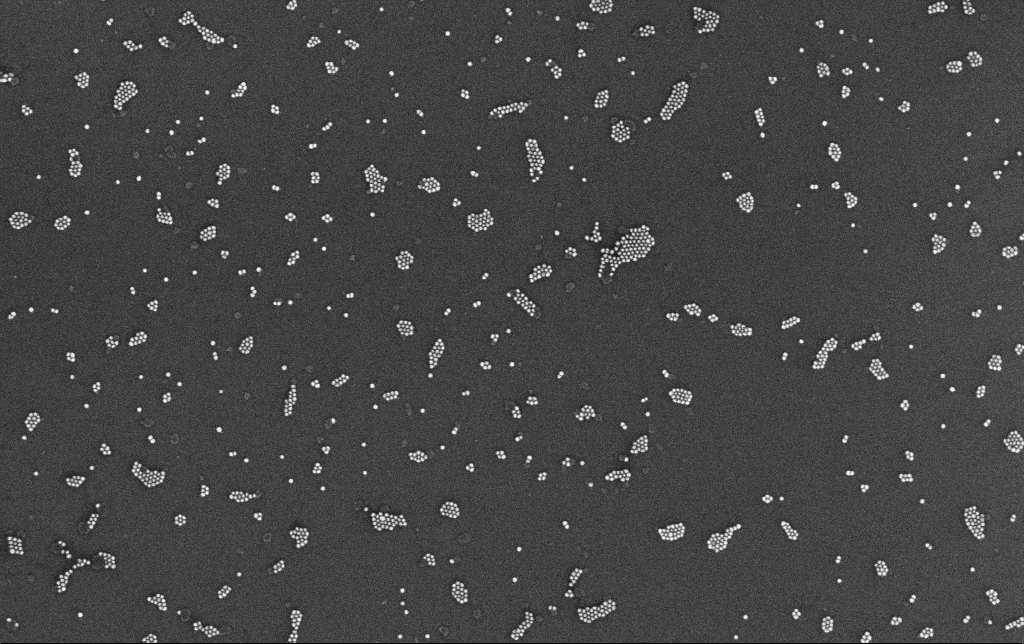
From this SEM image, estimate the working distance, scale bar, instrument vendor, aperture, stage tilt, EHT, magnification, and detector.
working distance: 3.4 mm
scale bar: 200 nm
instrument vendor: Zeiss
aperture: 30 µm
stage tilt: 0°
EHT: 10 kV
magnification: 100 K X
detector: InLens